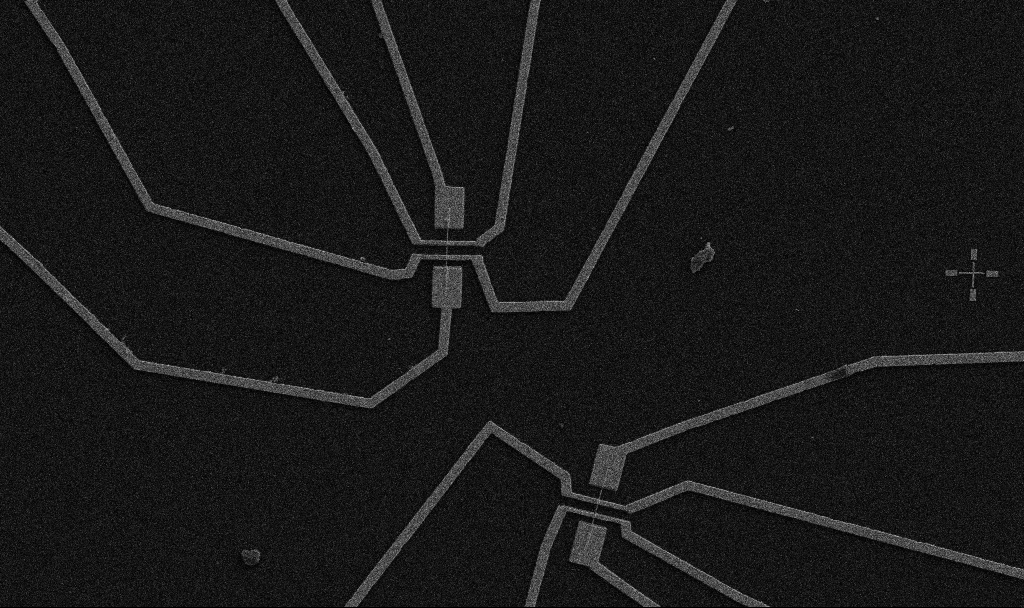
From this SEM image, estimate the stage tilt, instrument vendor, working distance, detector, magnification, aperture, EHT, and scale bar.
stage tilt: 0°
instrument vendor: Zeiss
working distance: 10.7 mm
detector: SE2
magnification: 5 K X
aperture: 30 µm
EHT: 5 kV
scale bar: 10000 nm